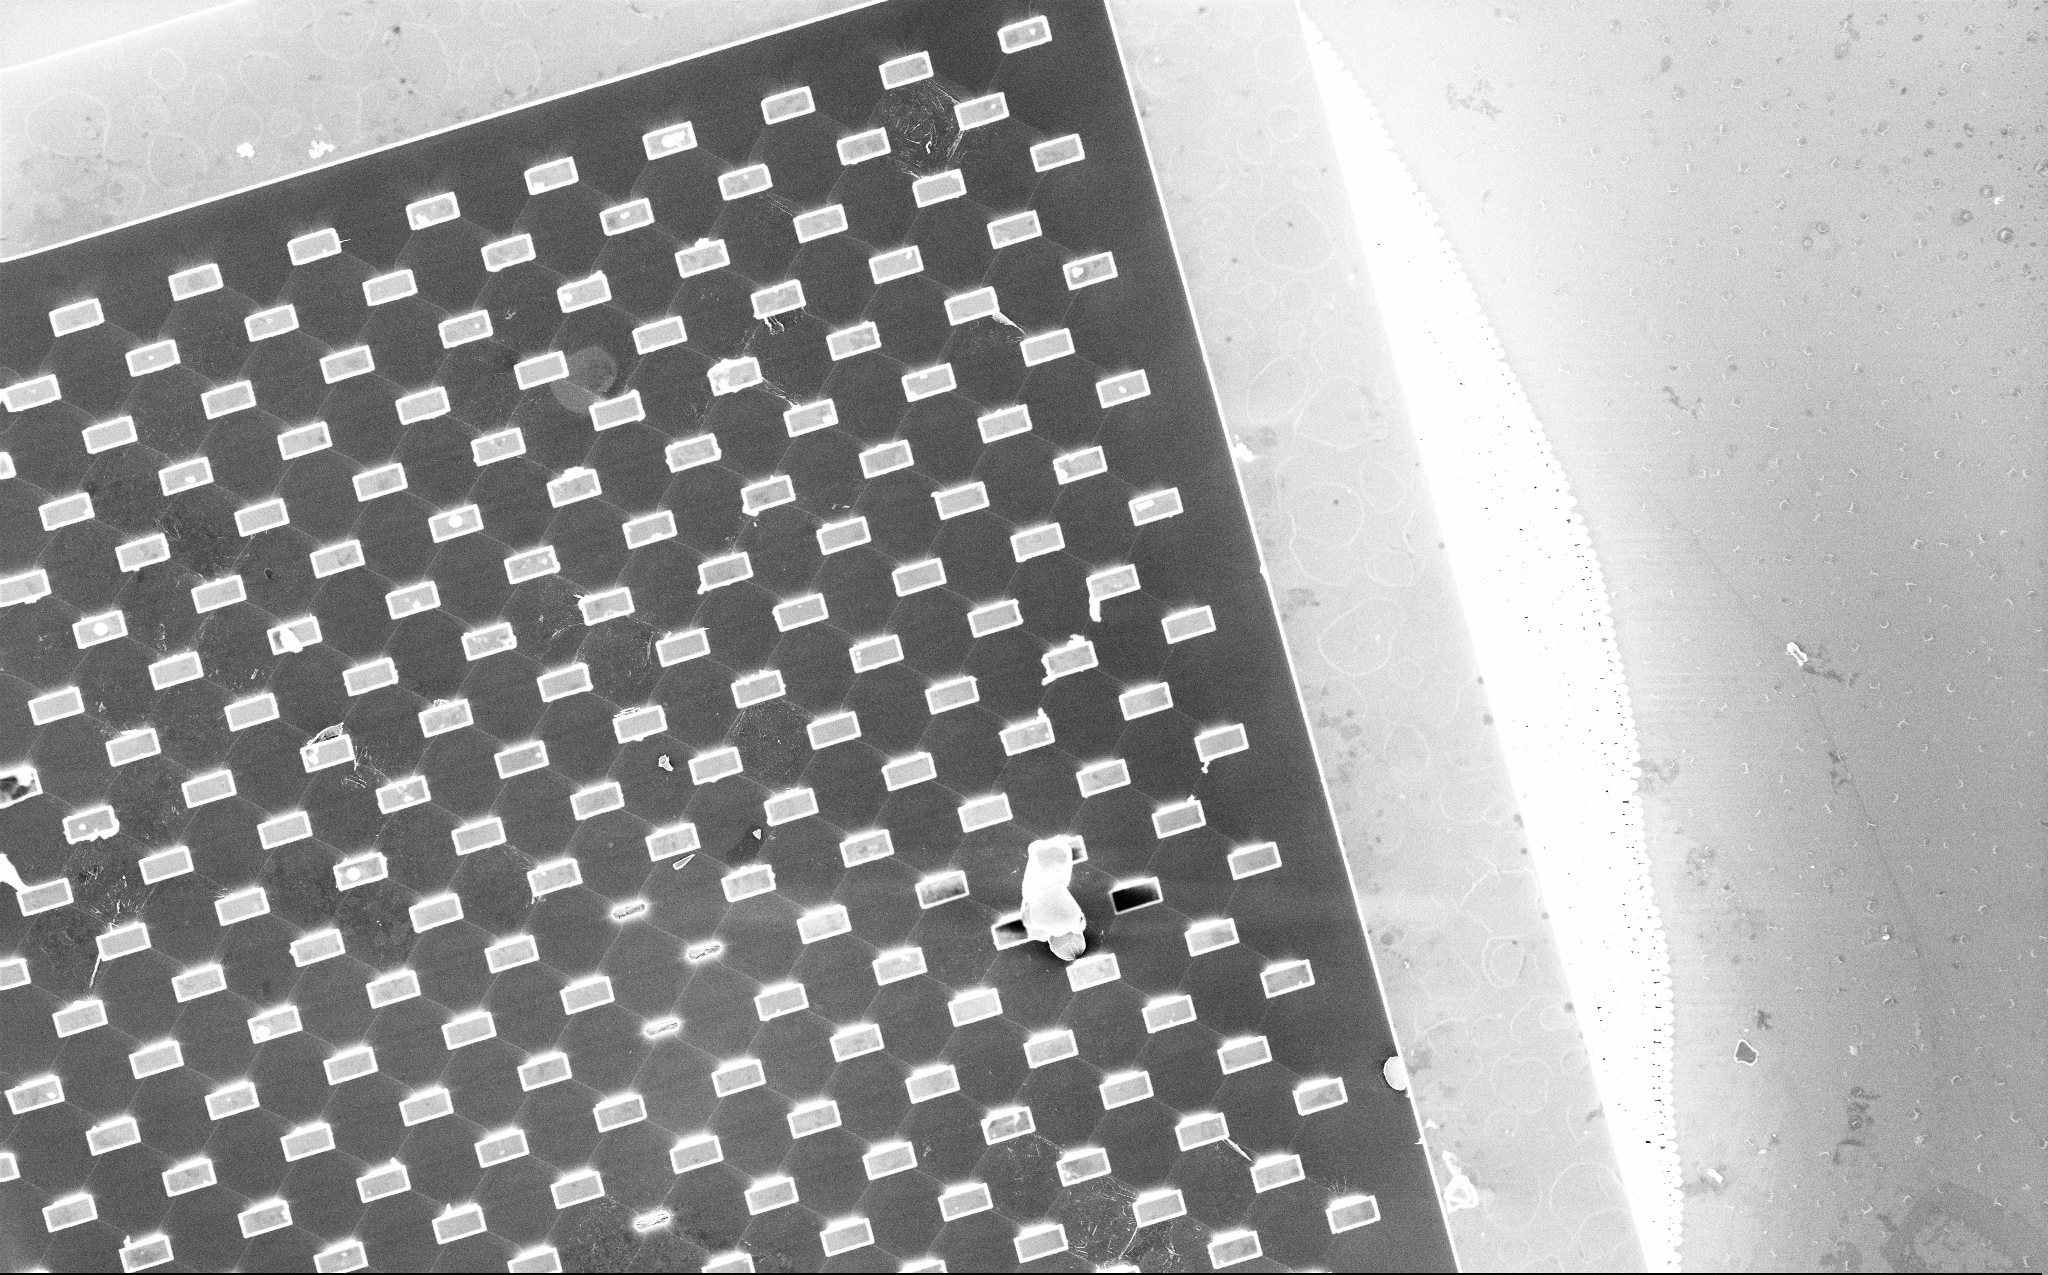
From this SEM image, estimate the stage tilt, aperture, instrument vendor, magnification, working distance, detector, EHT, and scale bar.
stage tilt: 0°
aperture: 30 µm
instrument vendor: Zeiss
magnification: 1.13 K X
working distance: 4 mm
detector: InLens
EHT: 5 kV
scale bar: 20000 nm